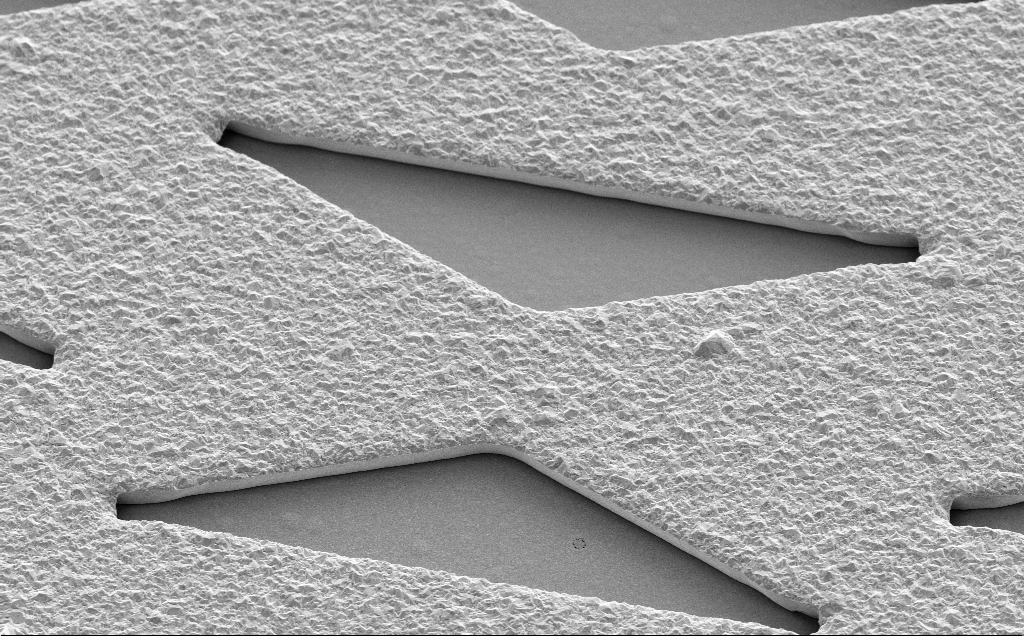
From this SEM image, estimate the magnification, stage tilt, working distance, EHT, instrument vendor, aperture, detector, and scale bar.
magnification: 7.03 K X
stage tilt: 35°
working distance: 13 mm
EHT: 5 kV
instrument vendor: Zeiss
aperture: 30 µm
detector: SE2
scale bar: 10000 nm